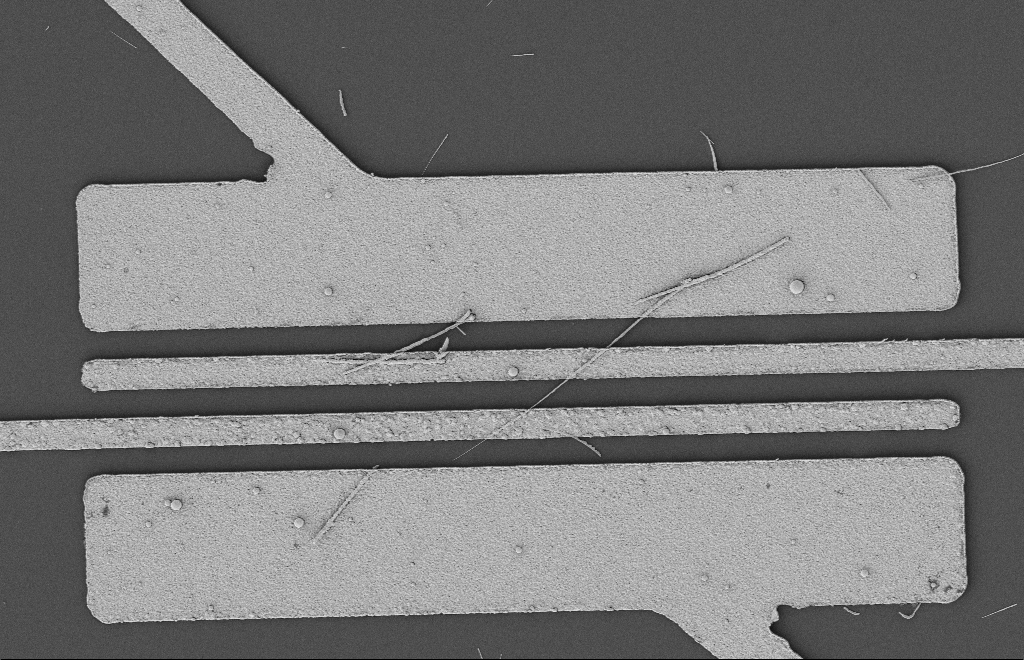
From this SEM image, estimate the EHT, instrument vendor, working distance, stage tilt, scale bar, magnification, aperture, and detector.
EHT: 2 kV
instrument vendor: Zeiss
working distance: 12 mm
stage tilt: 0°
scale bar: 2000 nm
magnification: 5.27 K X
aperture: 20 µm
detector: SE2